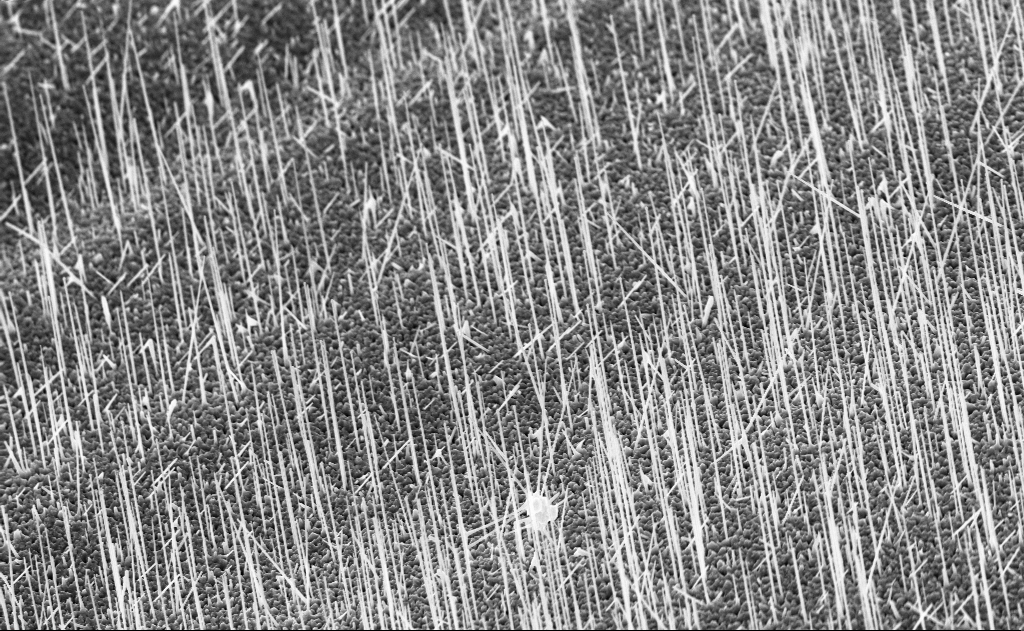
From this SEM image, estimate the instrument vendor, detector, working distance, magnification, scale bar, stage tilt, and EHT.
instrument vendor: Zeiss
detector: InLens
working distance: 8 mm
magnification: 10 K X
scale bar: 2000 nm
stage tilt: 0°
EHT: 10 kV